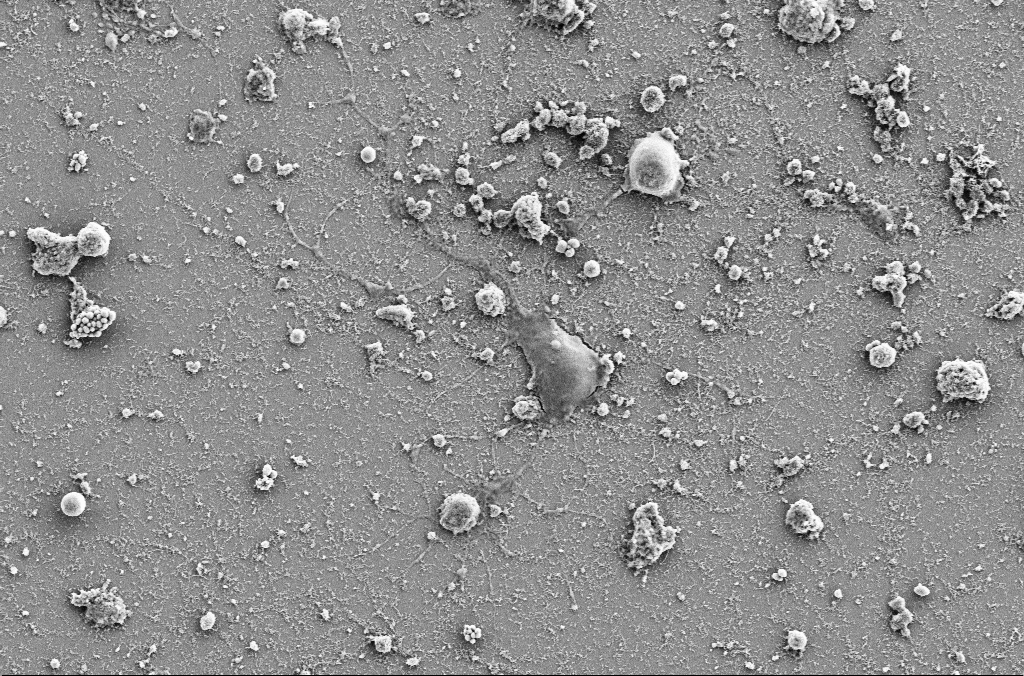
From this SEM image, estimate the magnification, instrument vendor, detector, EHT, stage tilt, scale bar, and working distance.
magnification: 3 K X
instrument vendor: Zeiss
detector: SE2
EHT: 5 kV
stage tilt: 0°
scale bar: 10000 nm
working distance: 4 mm